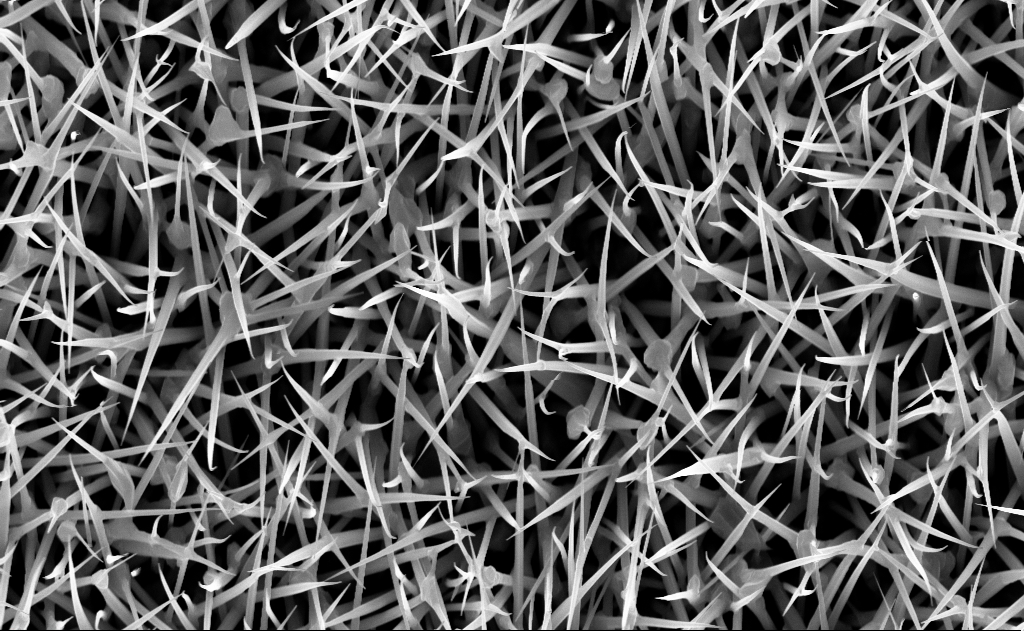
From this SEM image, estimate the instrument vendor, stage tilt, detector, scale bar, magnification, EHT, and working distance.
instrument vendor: Zeiss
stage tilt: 0°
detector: InLens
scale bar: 2000 nm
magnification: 20 K X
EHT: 10 kV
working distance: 7 mm